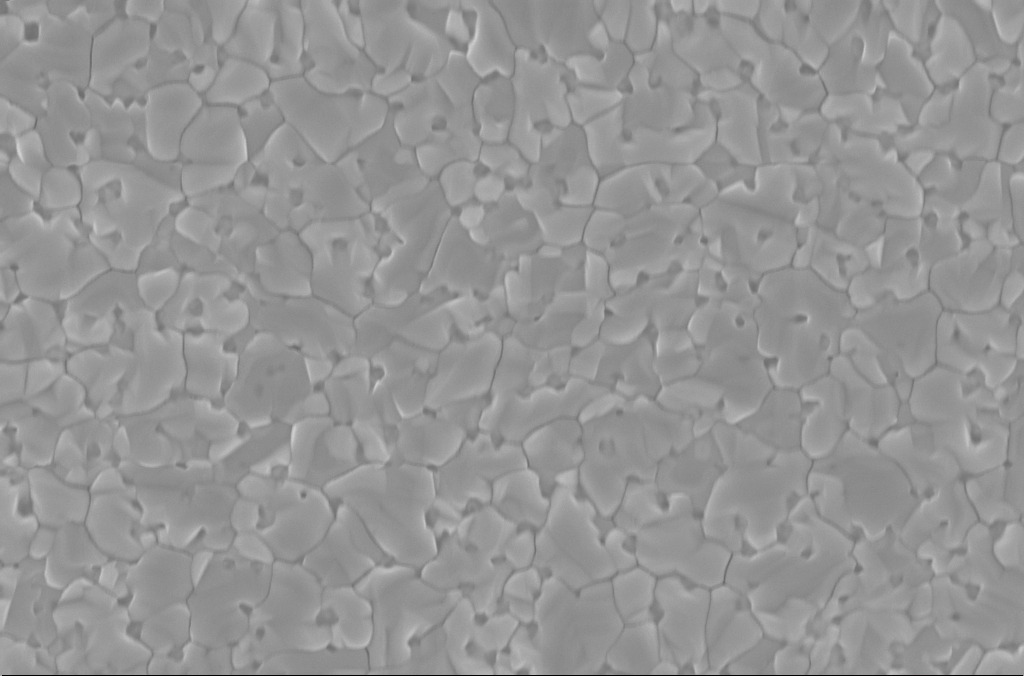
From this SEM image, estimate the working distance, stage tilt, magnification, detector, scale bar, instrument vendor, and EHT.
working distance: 3 mm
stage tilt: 0°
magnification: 40 K X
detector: InLens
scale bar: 1000 nm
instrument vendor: Zeiss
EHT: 5 kV